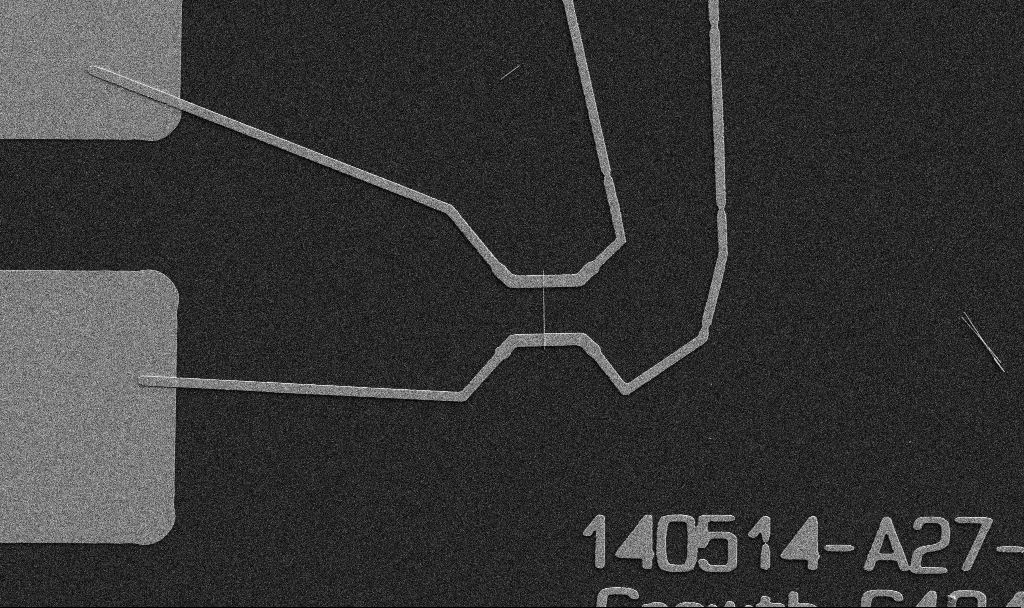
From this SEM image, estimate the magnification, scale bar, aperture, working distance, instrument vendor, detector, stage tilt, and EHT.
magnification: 5 K X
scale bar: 10000 nm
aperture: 30 µm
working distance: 10.7 mm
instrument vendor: Zeiss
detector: SE2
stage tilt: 0°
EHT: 5 kV